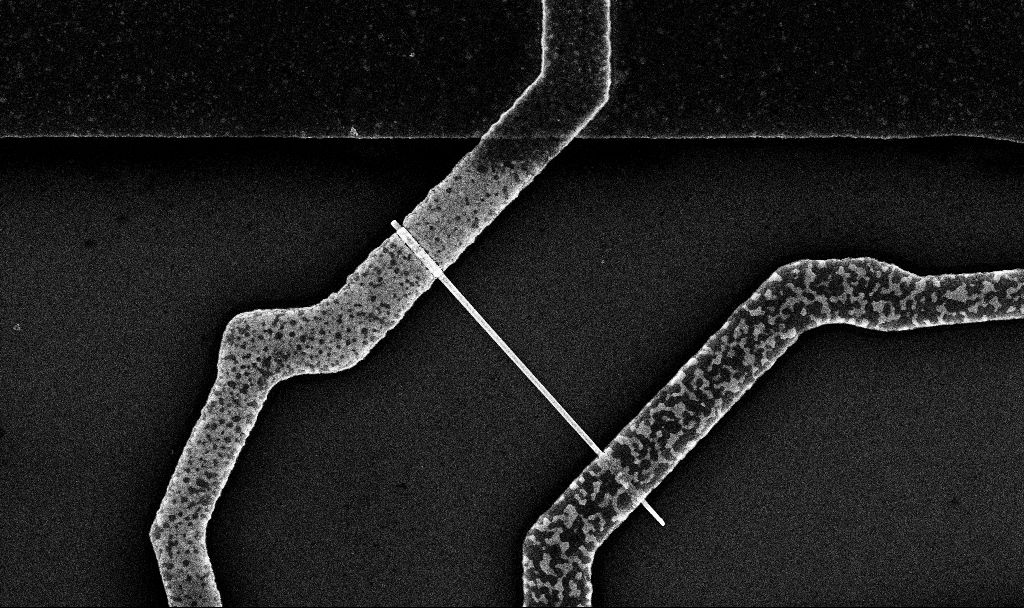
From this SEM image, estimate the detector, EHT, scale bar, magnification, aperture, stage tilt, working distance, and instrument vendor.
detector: InLens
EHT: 10 kV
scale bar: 1000 nm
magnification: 25.87 K X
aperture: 30 µm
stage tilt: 0°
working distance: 6.7 mm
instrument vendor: Zeiss